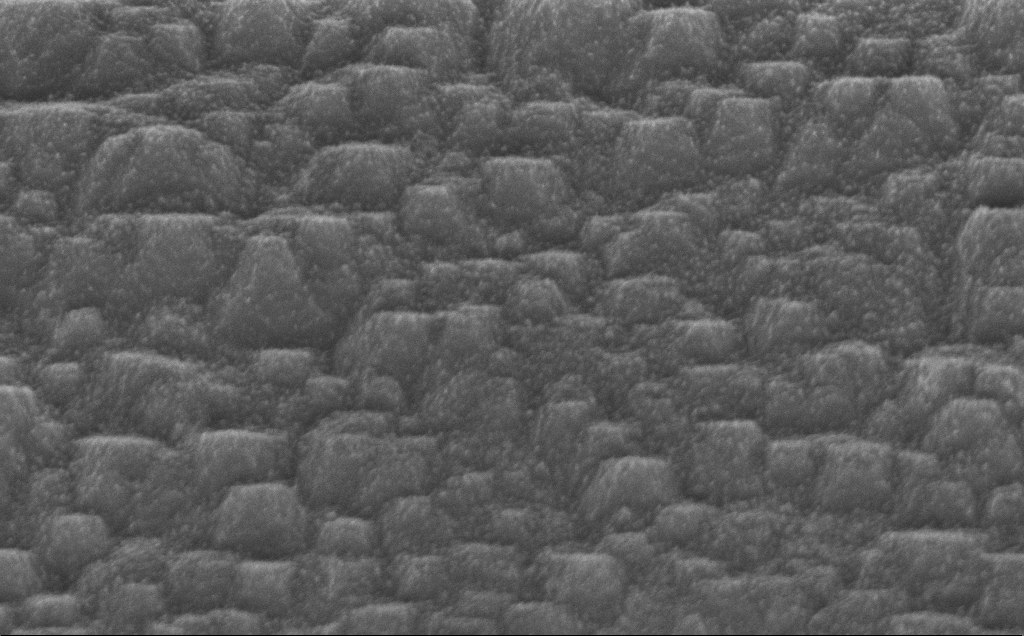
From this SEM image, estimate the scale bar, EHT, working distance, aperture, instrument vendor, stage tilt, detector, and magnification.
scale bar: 1000 nm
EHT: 5 kV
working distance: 13 mm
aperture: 30 µm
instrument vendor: Zeiss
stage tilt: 45°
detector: InLens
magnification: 43.23 K X